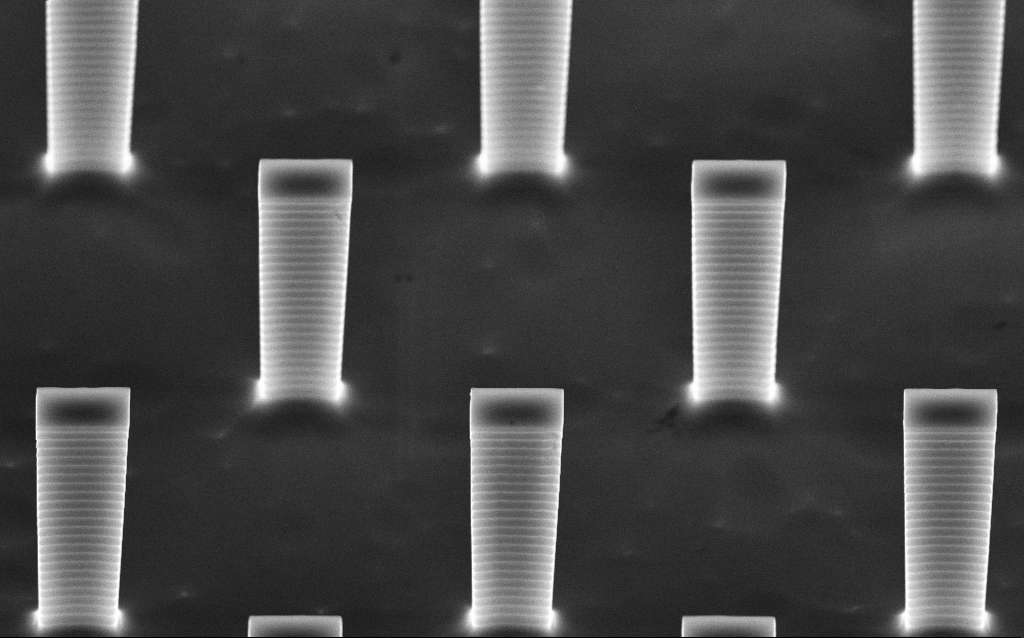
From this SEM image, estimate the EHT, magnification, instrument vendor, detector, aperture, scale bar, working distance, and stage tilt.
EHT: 10 kV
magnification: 13.26 K X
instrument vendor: Zeiss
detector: InLens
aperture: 30 µm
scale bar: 2000 nm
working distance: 5.1 mm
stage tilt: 45°